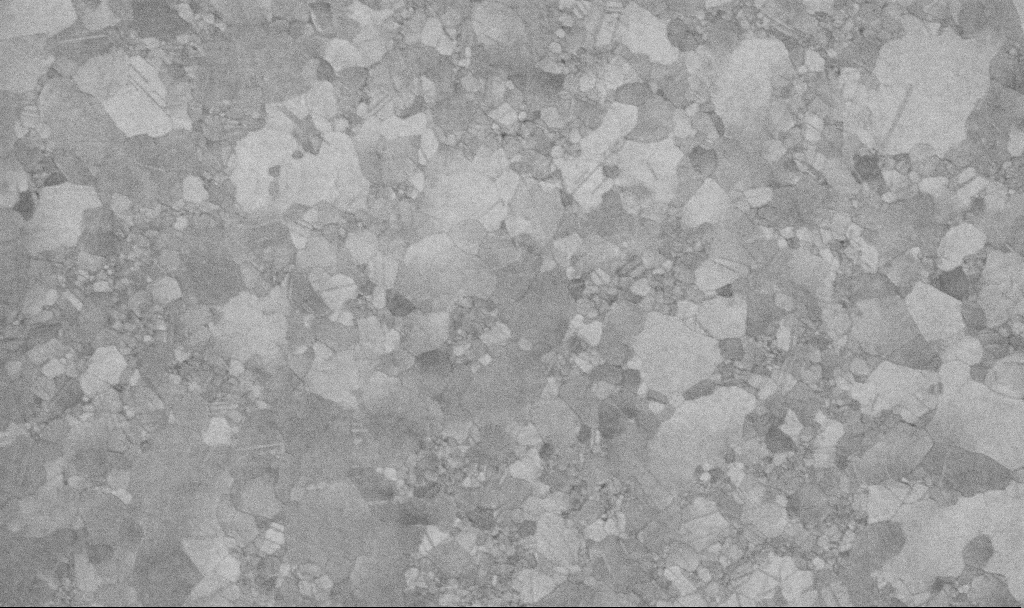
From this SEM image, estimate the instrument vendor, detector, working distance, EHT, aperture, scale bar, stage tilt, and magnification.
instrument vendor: Zeiss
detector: InLens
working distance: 3.4 mm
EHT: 10 kV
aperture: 30 µm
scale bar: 200 nm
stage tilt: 0°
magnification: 100 K X